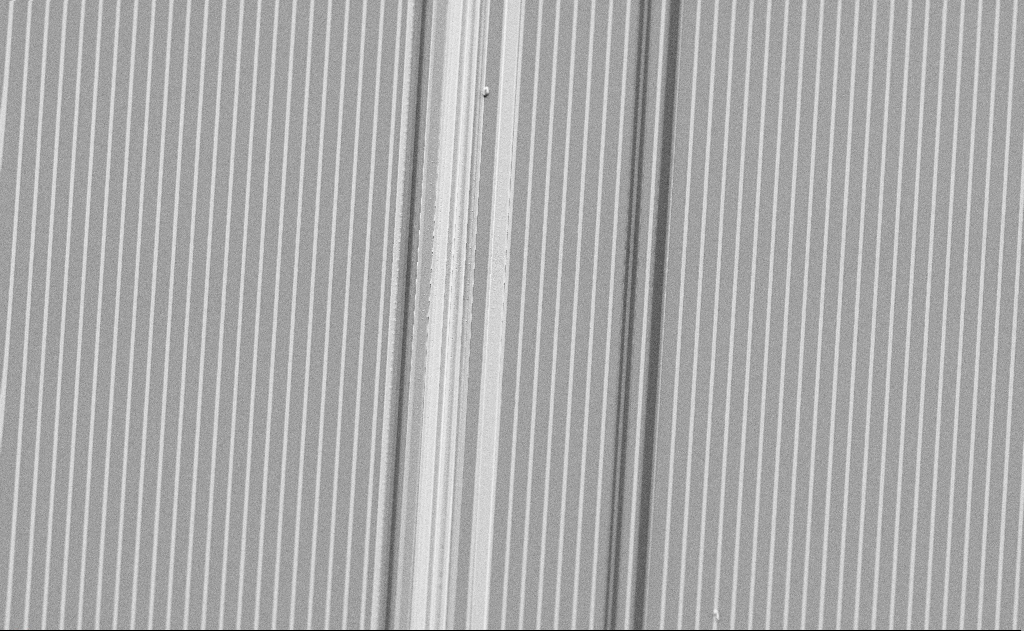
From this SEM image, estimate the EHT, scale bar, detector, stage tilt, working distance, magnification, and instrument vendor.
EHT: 6 kV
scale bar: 20000 nm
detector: SE2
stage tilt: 50°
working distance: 11 mm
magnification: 1.62 K X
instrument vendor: Zeiss